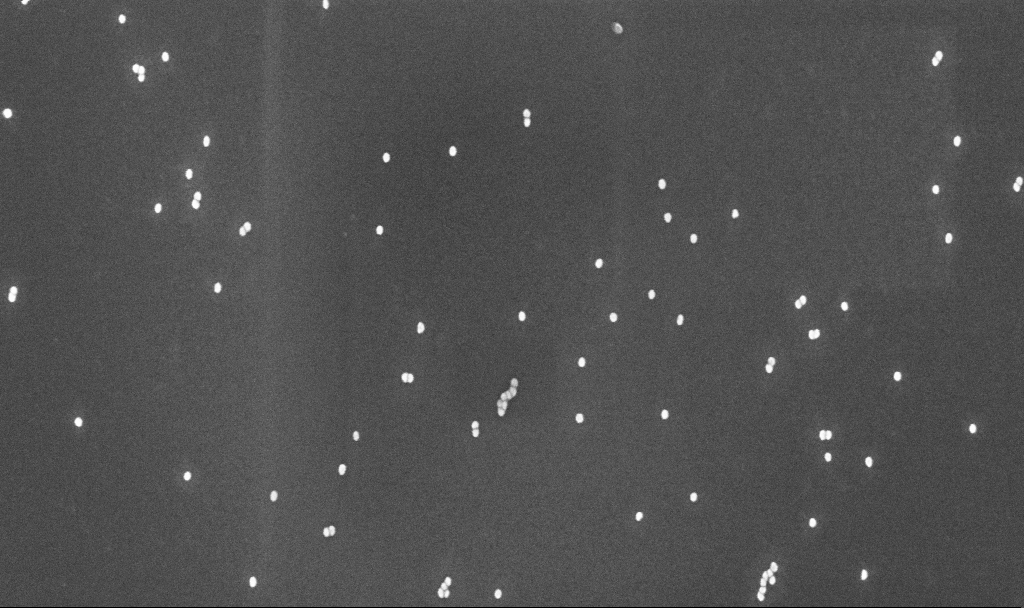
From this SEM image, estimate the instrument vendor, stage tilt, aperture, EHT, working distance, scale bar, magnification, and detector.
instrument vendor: Zeiss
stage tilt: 0°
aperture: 30 µm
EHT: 10 kV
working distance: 4.8 mm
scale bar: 200 nm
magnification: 100 K X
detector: InLens